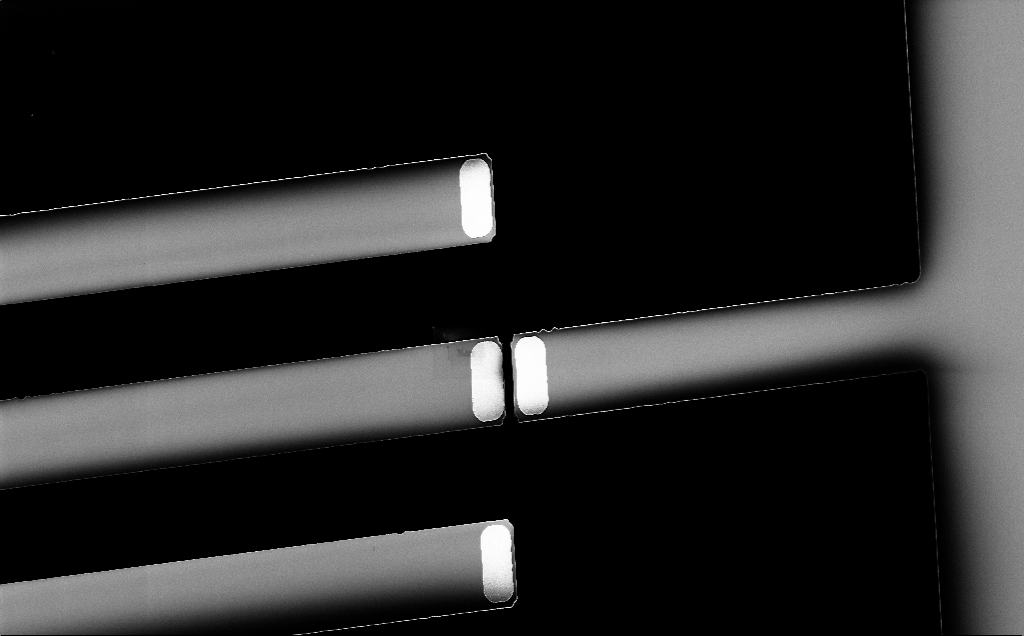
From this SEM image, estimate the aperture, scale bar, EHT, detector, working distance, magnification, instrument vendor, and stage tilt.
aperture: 30 µm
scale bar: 20000 nm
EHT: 5 kV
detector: InLens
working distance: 6 mm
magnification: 1.22 K X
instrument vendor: Zeiss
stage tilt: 0°